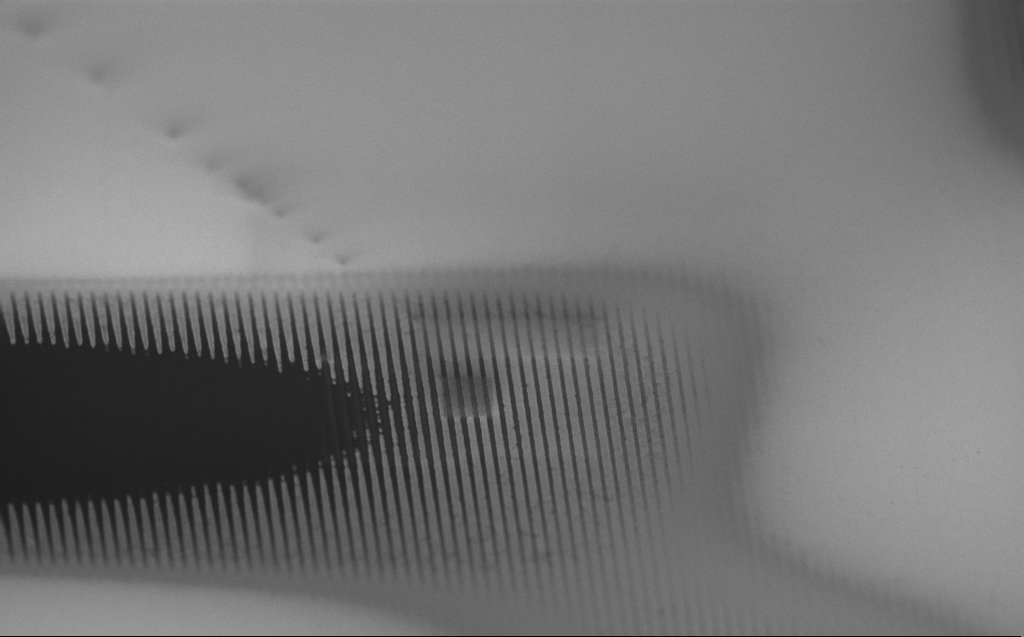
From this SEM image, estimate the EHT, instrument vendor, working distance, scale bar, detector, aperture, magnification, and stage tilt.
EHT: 1 kV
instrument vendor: Zeiss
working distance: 3 mm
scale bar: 2000 nm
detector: InLens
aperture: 30 µm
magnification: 9.77 K X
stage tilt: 60.7°